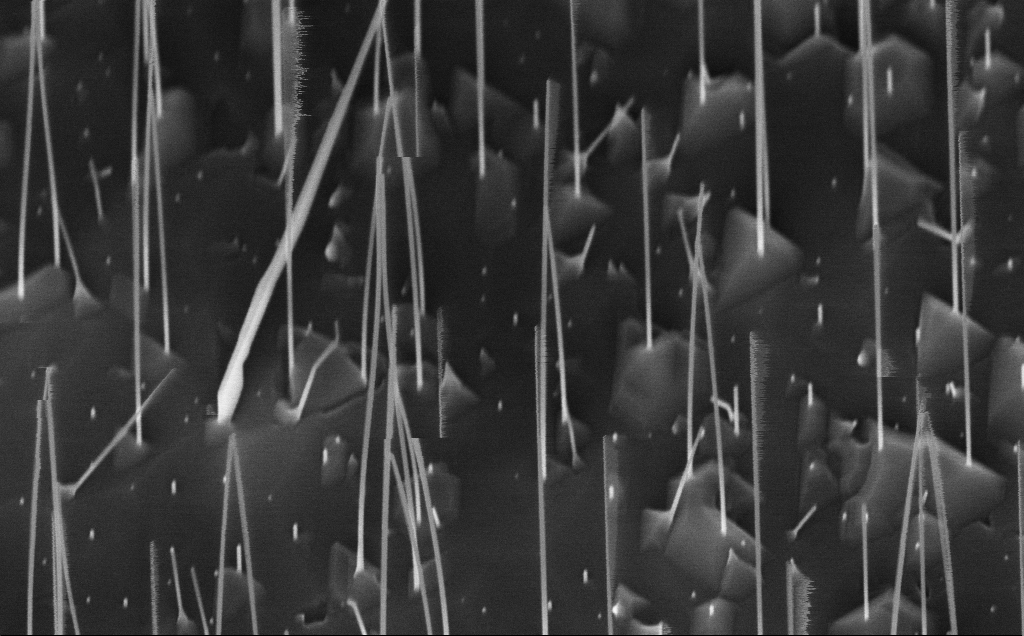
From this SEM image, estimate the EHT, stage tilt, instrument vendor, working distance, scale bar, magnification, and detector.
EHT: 10 kV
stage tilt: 45°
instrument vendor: Zeiss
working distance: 7 mm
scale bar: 1000 nm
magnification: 58.73 K X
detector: InLens